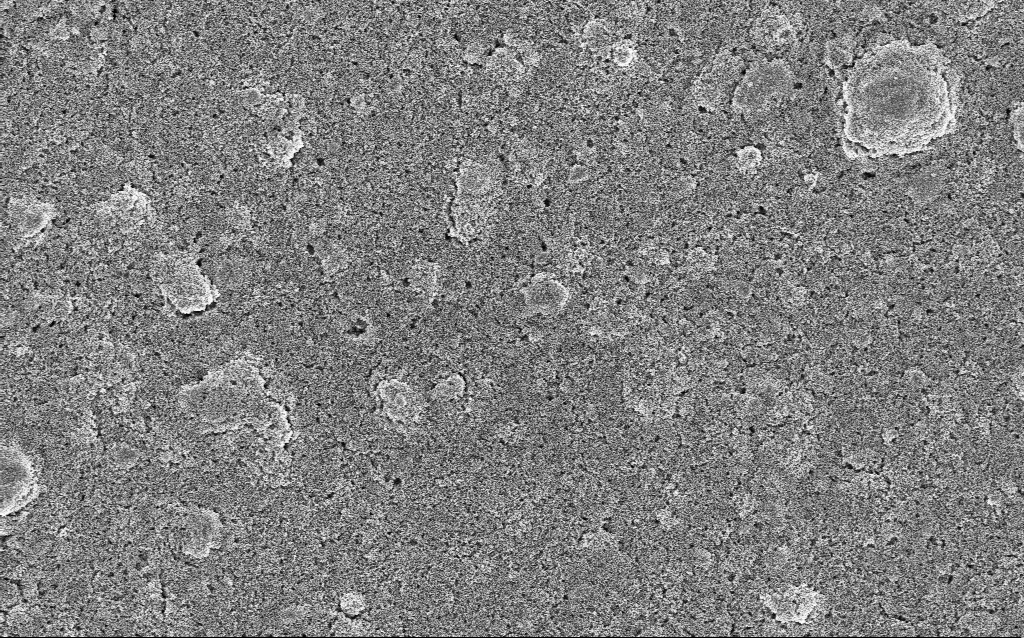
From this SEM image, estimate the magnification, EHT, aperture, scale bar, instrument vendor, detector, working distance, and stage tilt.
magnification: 5 K X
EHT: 5 kV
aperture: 30 µm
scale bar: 10000 nm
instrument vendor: Zeiss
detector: InLens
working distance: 5.3 mm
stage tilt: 0°